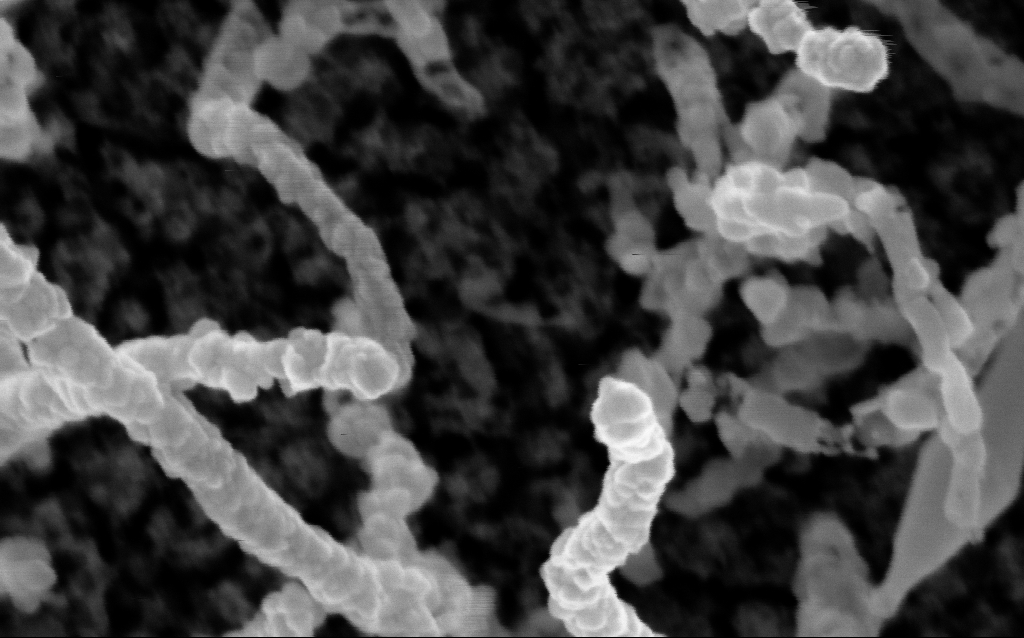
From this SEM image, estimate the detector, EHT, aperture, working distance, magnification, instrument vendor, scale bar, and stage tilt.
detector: InLens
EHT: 5 kV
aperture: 30 µm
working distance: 2.2 mm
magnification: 364.55 K X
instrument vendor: Zeiss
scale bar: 100 nm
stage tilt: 0°